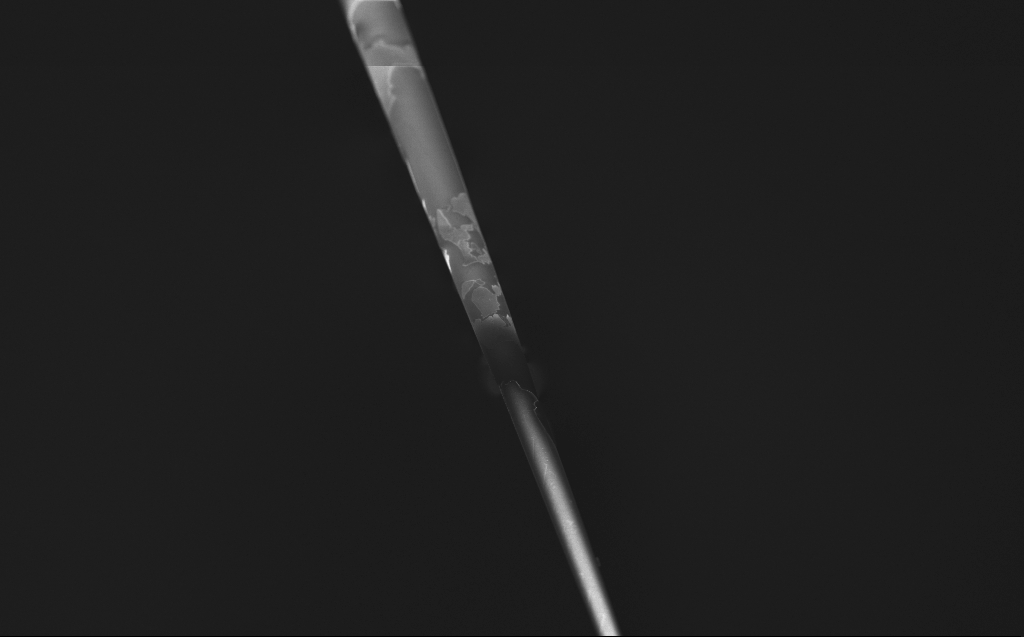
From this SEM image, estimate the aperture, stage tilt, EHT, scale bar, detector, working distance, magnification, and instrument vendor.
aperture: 30 µm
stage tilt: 45°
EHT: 1 kV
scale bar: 100000 nm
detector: InLens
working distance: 4 mm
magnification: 0.5 K X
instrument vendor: Zeiss